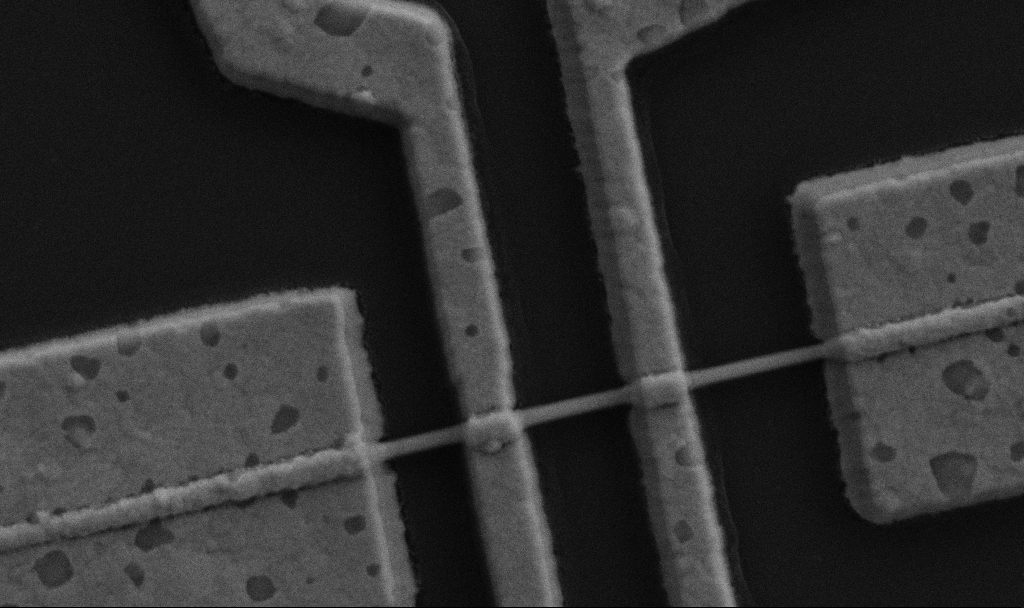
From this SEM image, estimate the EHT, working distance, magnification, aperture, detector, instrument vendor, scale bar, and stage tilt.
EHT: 5 kV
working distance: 9.7 mm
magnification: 60 K X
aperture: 30 µm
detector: SE2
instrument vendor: Zeiss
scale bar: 1000 nm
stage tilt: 0°